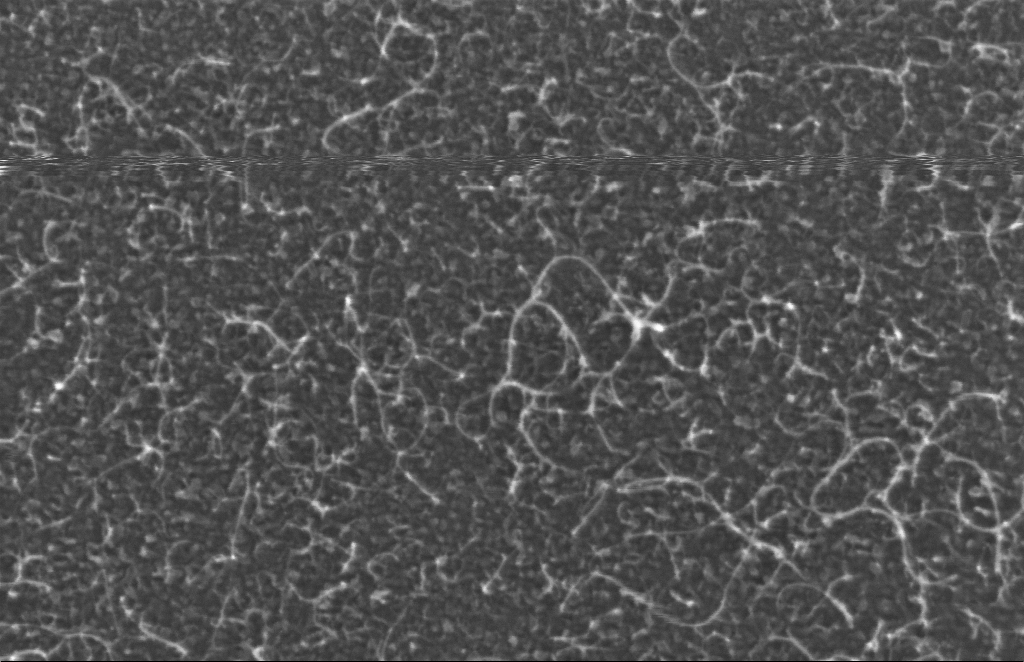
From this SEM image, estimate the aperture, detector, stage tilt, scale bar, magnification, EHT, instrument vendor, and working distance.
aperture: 30 µm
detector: InLens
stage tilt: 0°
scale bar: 100 nm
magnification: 338.3 K X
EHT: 5 kV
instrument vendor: Zeiss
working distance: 4 mm